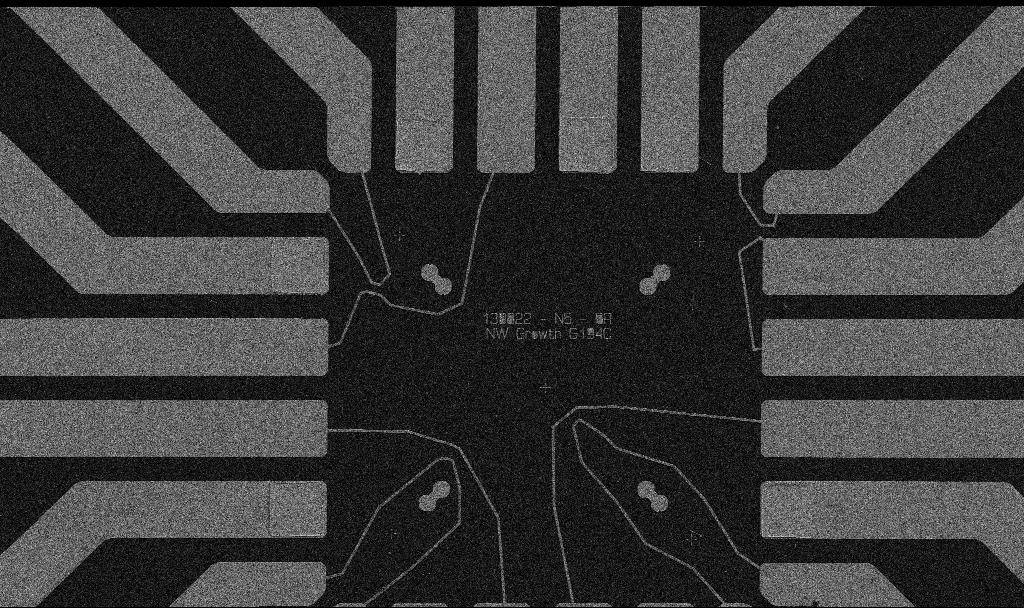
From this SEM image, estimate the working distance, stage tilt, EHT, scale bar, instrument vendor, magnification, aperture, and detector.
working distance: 10.7 mm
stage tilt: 0°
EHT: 5 kV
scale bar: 20000 nm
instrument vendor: Zeiss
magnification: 1 K X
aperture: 30 µm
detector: SE2